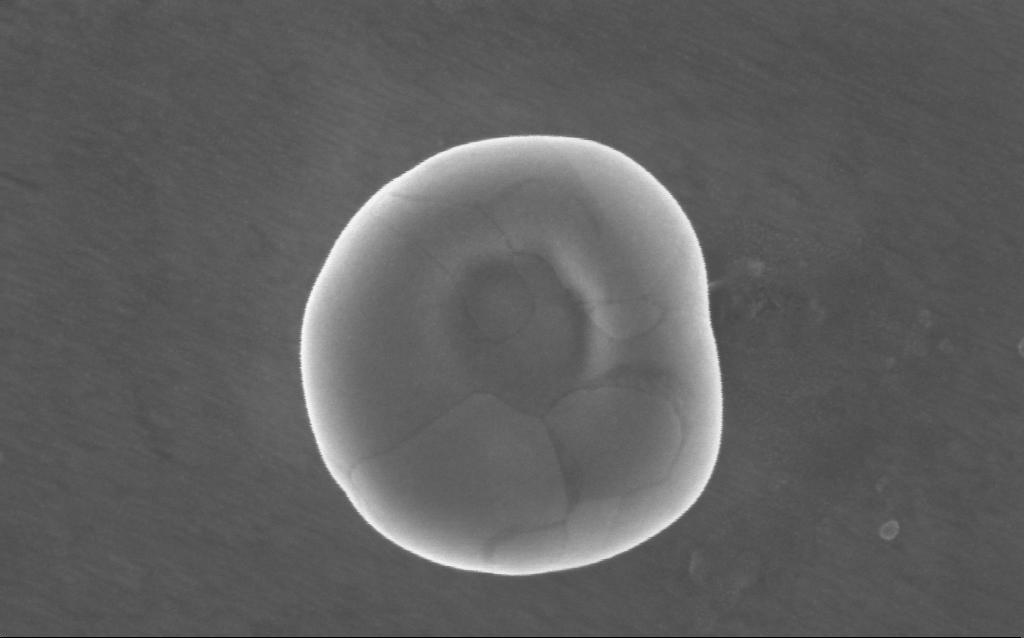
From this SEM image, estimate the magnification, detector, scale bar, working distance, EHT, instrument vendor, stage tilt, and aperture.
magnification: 232 K X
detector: InLens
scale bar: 200 nm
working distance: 3 mm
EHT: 5 kV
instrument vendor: Zeiss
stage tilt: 0°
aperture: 30 µm